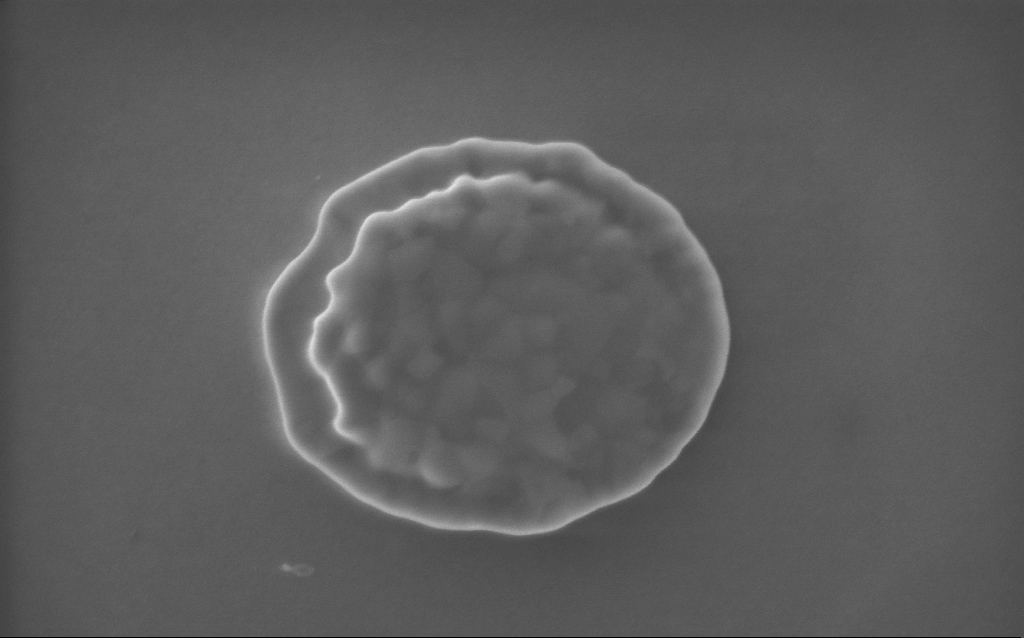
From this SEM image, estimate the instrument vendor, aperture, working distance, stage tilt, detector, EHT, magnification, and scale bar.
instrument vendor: Zeiss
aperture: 30 µm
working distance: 2 mm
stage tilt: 0°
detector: InLens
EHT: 5 kV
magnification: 88.38 K X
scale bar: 200 nm